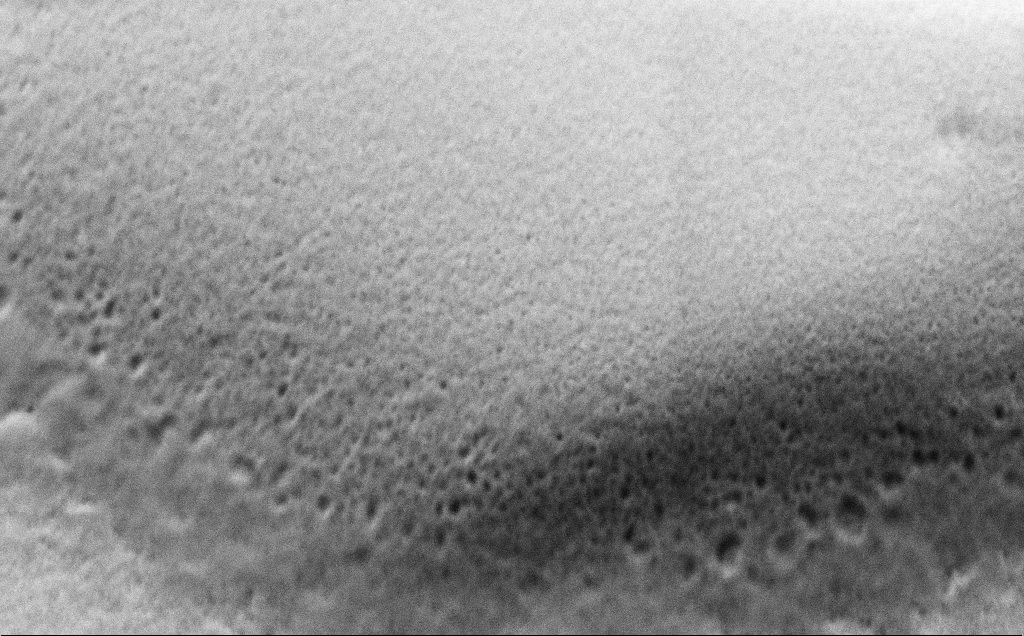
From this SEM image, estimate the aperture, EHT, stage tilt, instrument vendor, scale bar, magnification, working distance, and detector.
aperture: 30 µm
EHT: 1.5 kV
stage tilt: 45°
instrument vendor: Zeiss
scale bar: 1000 nm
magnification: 57.32 K X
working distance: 4 mm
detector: SE2